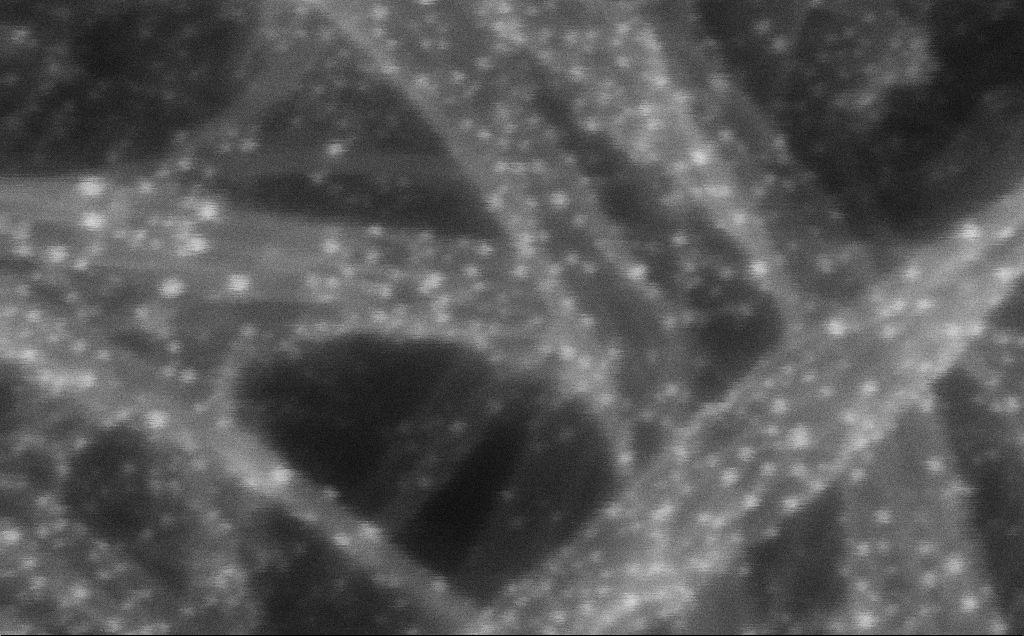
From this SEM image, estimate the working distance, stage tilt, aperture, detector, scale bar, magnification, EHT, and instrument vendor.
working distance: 3 mm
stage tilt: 0°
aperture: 30 µm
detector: InLens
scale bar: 20 nm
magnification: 1031.08 K X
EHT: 10 kV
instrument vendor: Zeiss